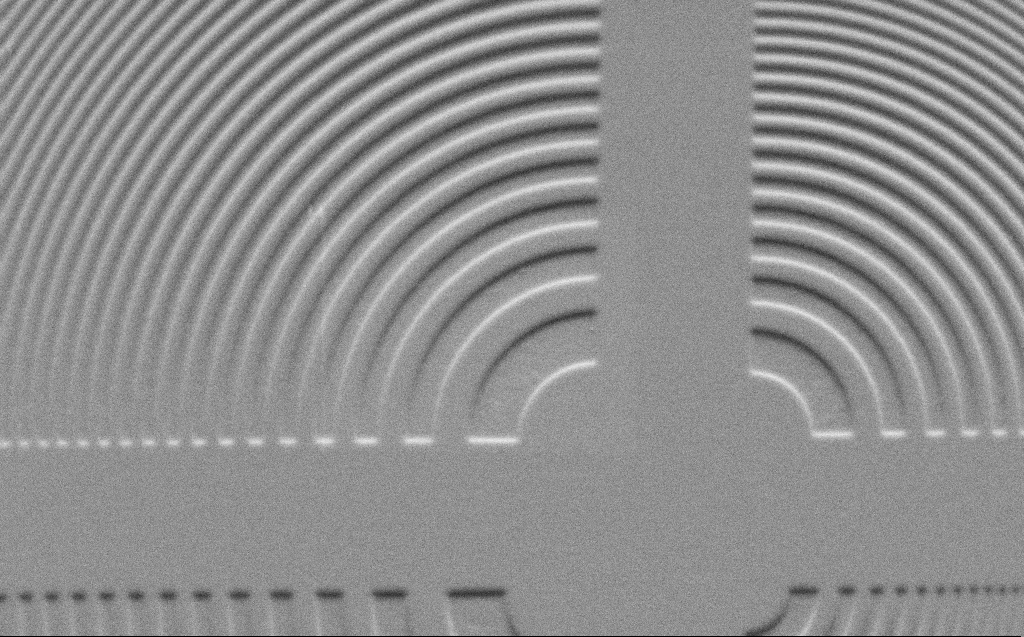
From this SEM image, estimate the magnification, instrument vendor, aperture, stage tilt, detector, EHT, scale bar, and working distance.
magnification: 5.72 K X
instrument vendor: Zeiss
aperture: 30 µm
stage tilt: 45°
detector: SE2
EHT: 1 kV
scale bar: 10000 nm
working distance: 5 mm